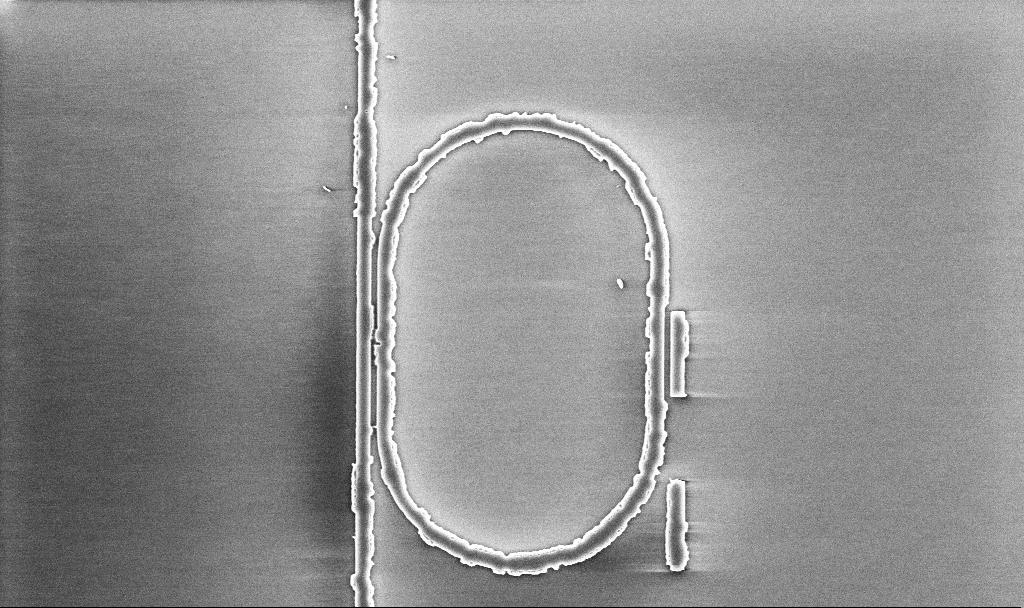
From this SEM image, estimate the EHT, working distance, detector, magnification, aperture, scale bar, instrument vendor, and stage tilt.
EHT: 5 kV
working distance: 10.1 mm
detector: InLens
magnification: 10.62 K X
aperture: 30 µm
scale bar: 2000 nm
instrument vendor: Zeiss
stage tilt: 0°